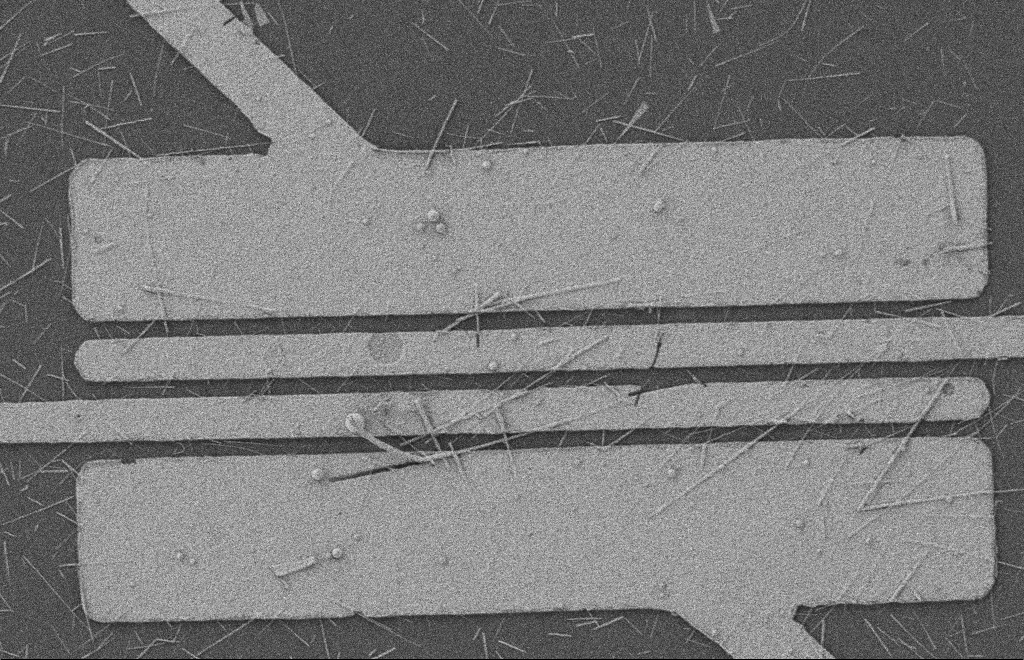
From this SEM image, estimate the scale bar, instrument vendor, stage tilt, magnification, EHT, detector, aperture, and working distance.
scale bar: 2000 nm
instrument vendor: Zeiss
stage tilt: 0°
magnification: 5.5 K X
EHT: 2 kV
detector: SE2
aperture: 20 µm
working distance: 8 mm